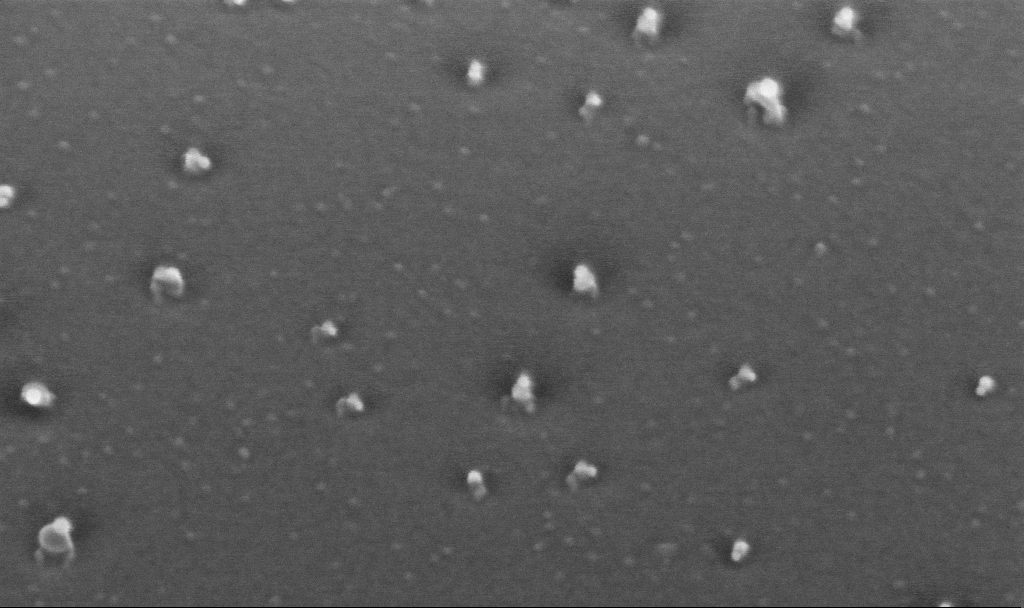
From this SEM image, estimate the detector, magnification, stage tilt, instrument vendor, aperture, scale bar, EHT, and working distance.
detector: InLens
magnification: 200 K X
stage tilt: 45°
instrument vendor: Zeiss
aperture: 30 µm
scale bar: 200 nm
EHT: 10 kV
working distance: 4.4 mm